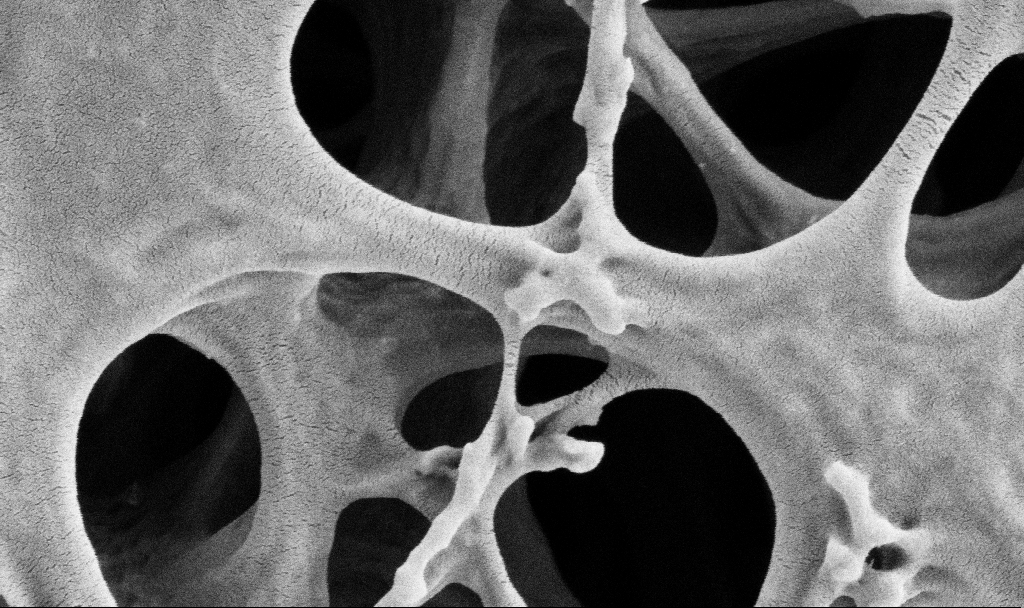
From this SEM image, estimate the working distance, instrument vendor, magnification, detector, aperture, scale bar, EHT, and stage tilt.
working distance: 3.5 mm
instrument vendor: Zeiss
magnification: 100 K X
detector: SE2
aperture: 30 µm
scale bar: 200 nm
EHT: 2 kV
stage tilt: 0°